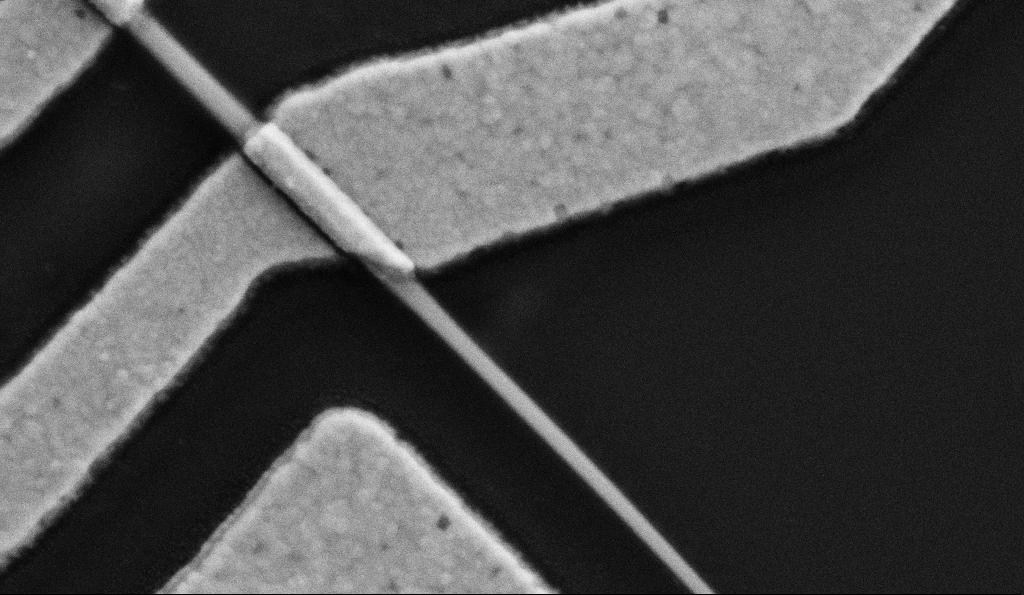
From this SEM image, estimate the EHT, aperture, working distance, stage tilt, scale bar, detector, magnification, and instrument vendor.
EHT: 5 kV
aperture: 30 µm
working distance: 9.6 mm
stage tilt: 0°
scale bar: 200 nm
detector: SE2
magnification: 100 K X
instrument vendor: Zeiss